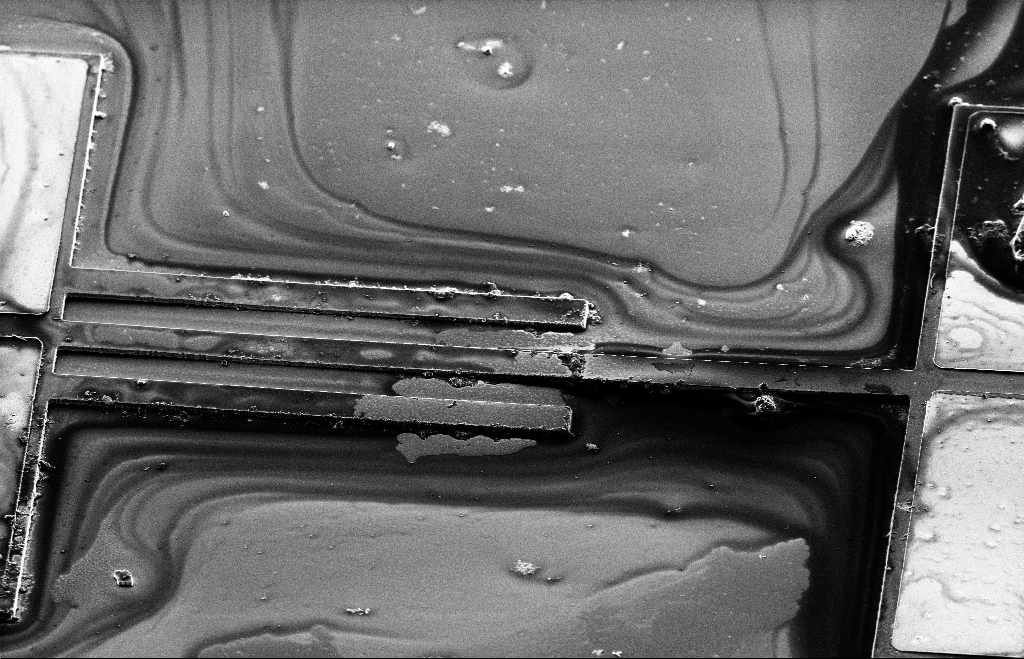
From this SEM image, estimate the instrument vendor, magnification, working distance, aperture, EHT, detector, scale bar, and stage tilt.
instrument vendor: Zeiss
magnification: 0.699 K X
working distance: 9 mm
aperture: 20 µm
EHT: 5 kV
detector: SE2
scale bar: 20000 nm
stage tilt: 40.8°